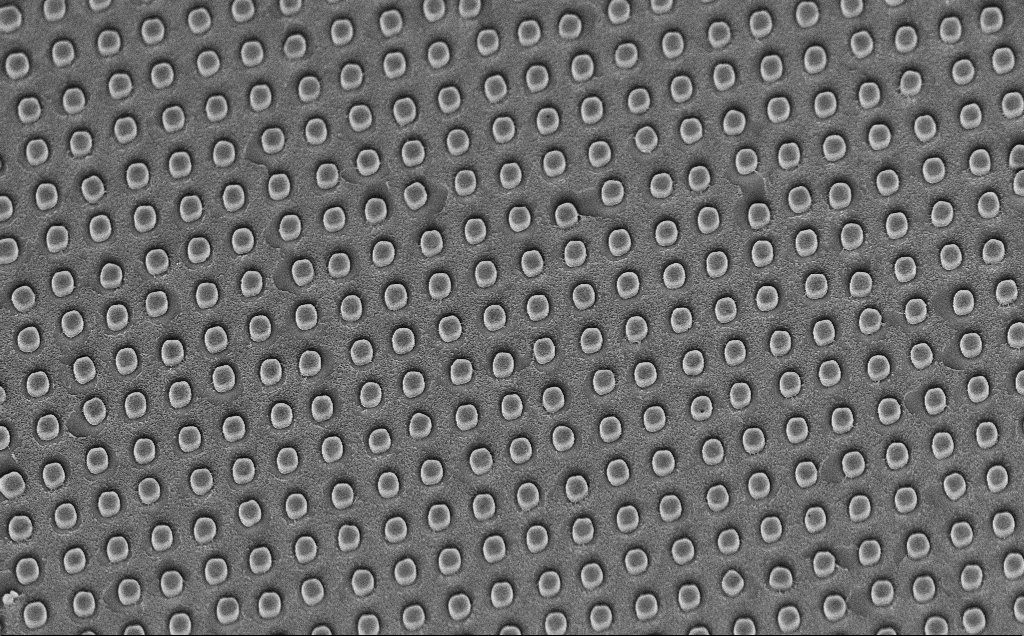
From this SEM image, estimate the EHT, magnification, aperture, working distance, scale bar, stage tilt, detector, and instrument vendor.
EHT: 5 kV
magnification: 11.23 K X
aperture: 30 µm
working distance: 7 mm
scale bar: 2000 nm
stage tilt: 45°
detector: InLens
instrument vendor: Zeiss